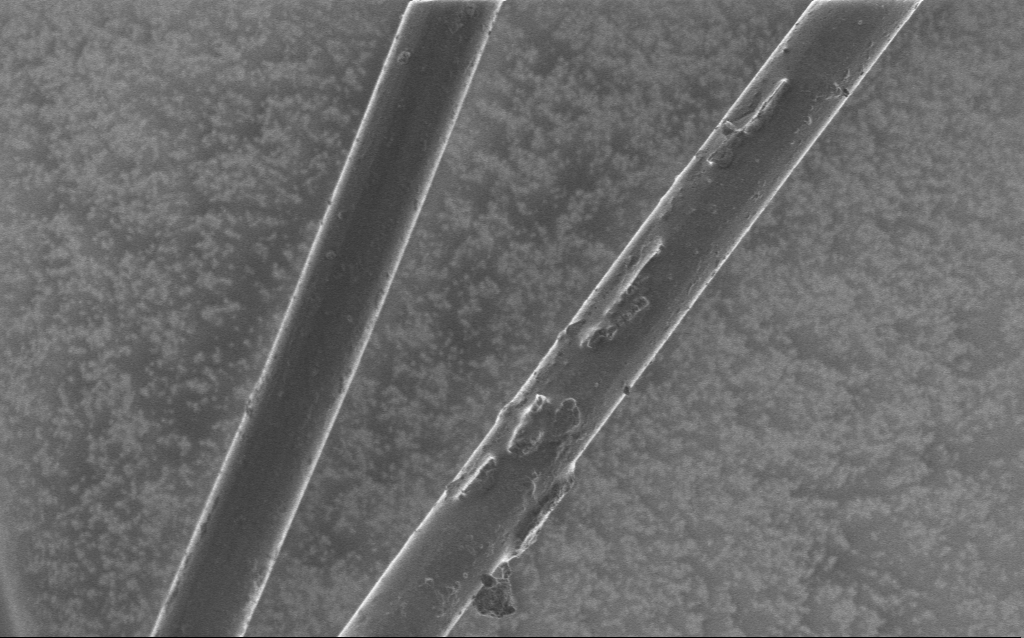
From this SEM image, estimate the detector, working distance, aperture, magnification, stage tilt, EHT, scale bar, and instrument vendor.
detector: InLens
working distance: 5 mm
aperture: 30 µm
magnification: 1.4 K X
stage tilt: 0°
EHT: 1 kV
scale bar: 20000 nm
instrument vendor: Zeiss